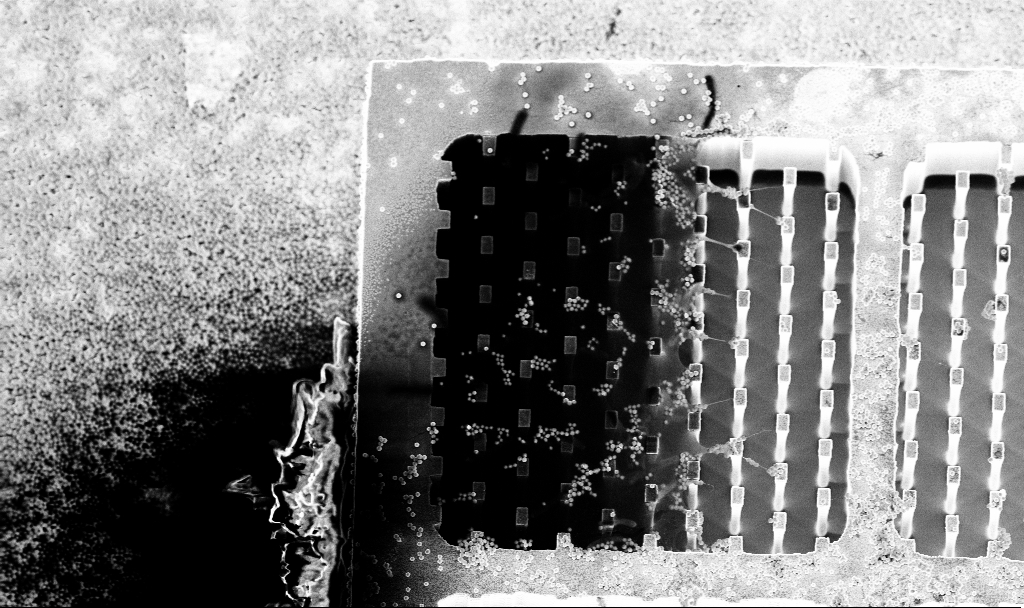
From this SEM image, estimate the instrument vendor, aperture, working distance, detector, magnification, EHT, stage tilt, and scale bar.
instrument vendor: Zeiss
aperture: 30 µm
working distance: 3.2 mm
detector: InLens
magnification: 2.9 K X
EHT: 5 kV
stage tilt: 15°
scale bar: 10000 nm